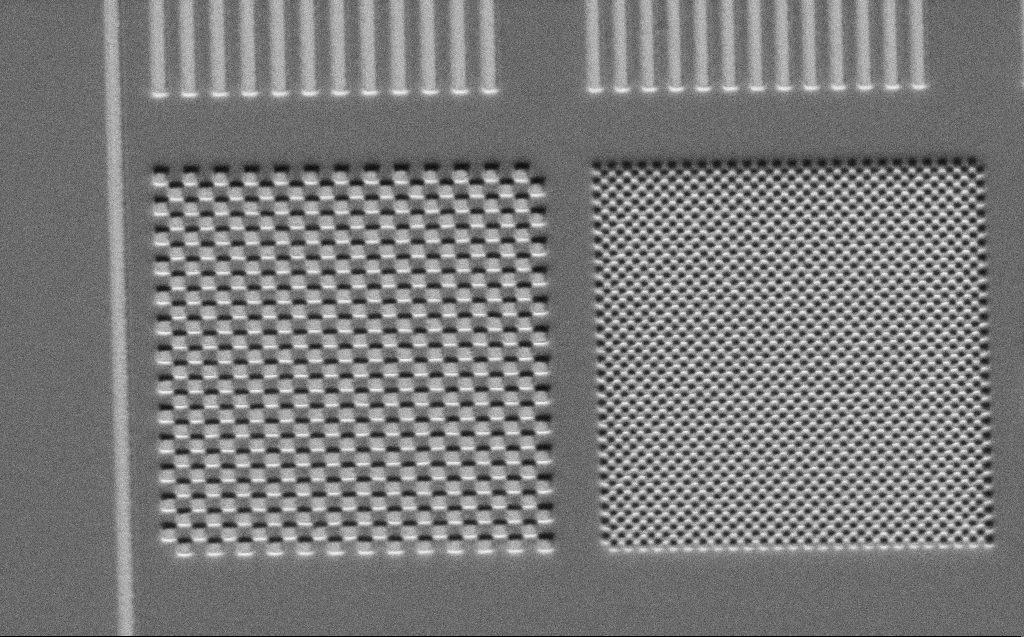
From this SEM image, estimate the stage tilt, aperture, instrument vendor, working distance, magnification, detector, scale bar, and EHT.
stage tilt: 45°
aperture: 30 µm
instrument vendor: Zeiss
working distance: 6 mm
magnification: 5.56 K X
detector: SE2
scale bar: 10000 nm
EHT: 3 kV